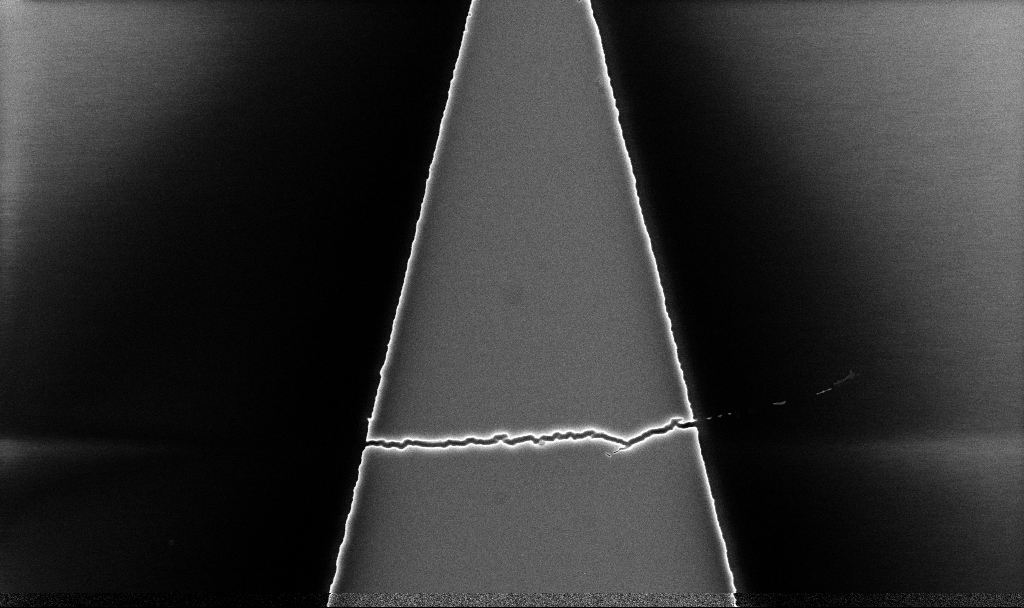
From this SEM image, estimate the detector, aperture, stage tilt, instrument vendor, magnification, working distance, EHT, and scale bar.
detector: InLens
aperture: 30 µm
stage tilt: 0°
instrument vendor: Zeiss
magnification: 13.08 K X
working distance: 5.2 mm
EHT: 5 kV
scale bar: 2000 nm